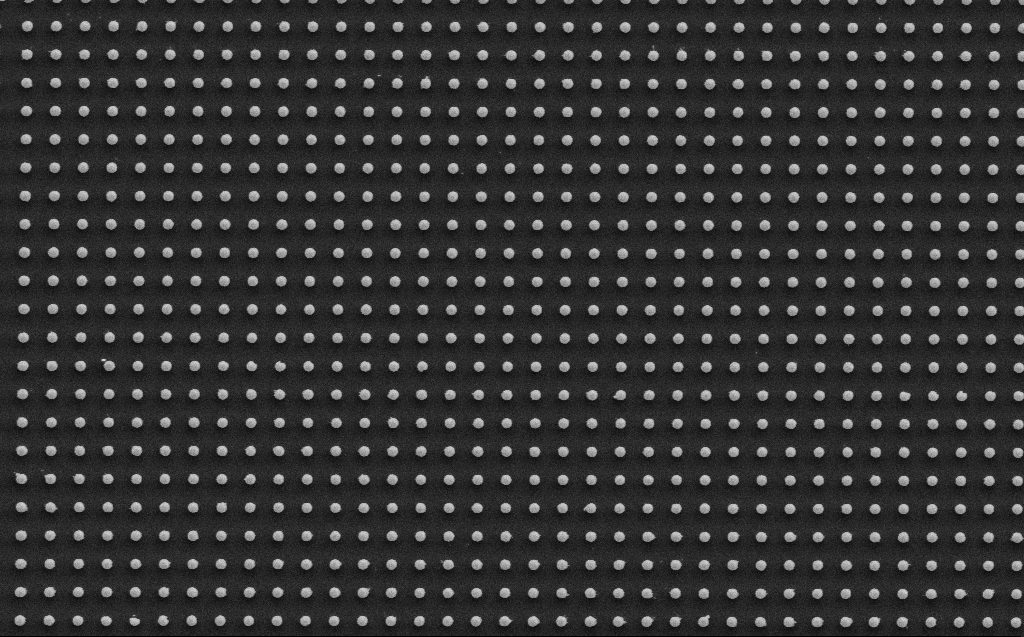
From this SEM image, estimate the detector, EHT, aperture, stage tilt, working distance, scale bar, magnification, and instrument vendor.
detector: SE2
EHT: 5 kV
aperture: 30 µm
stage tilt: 0°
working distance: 6 mm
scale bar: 2000 nm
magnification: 10 K X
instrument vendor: Zeiss